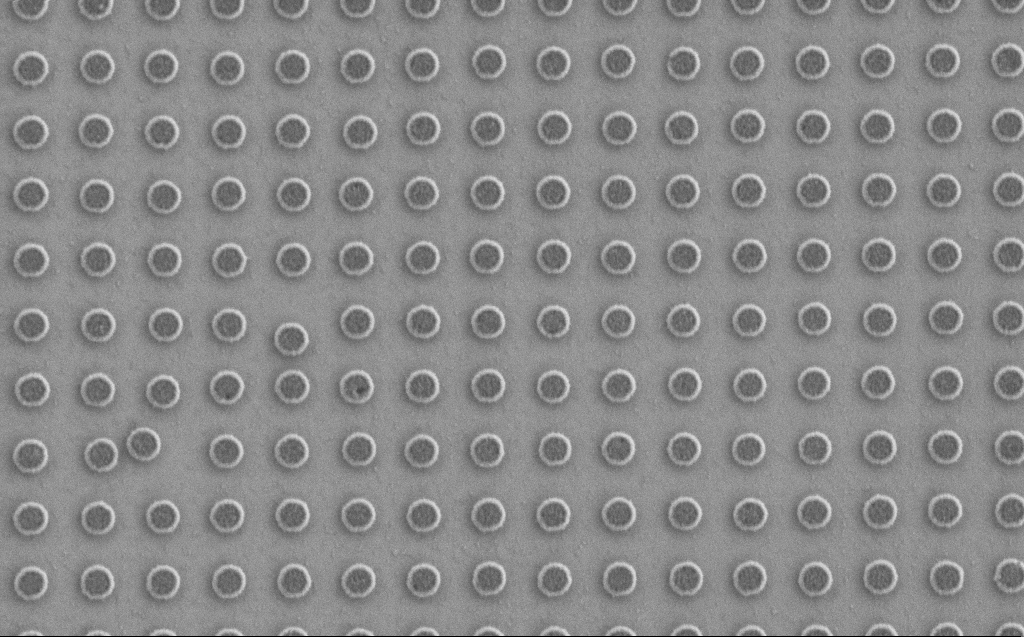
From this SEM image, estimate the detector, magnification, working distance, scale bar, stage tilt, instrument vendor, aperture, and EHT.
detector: SE2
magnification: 29.87 K X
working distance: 5 mm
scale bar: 2000 nm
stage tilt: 0°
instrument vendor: Zeiss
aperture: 30 µm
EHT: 1.2 kV